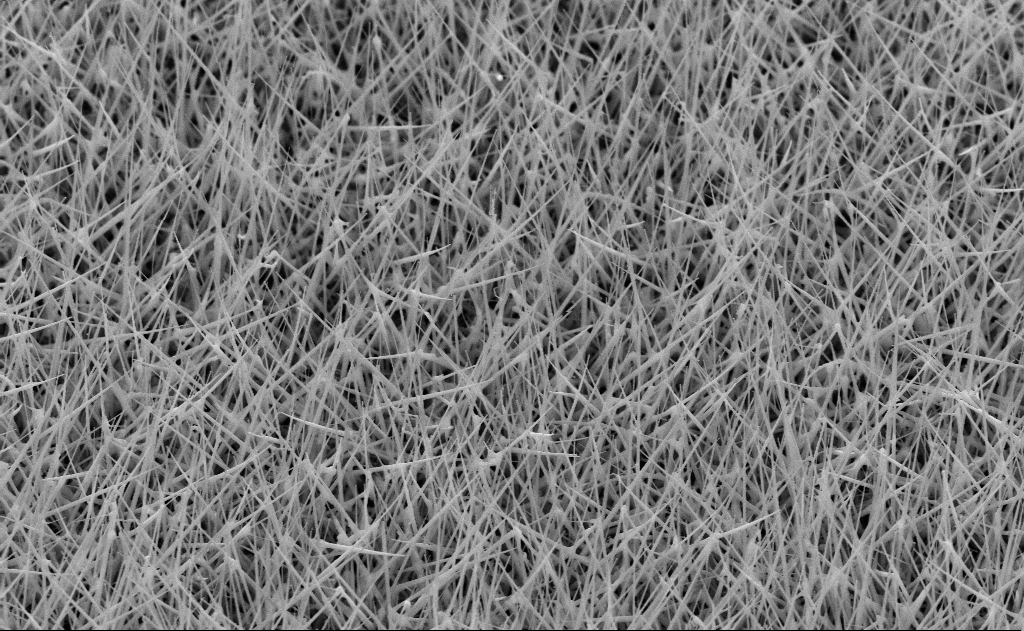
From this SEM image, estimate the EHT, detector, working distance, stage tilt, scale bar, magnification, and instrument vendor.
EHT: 10 kV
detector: SE2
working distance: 11 mm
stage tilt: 45°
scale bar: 2000 nm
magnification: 20 K X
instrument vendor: Zeiss